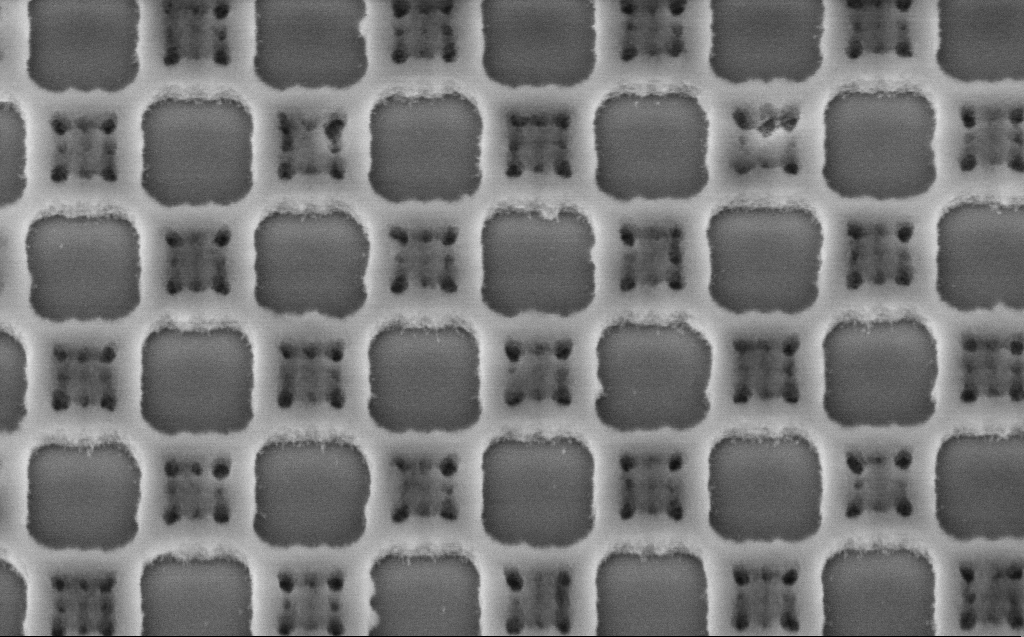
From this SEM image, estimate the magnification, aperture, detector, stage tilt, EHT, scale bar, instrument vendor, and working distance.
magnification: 84.15 K X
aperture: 30 µm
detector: InLens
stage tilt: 30°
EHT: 3 kV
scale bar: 200 nm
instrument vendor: Zeiss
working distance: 6 mm